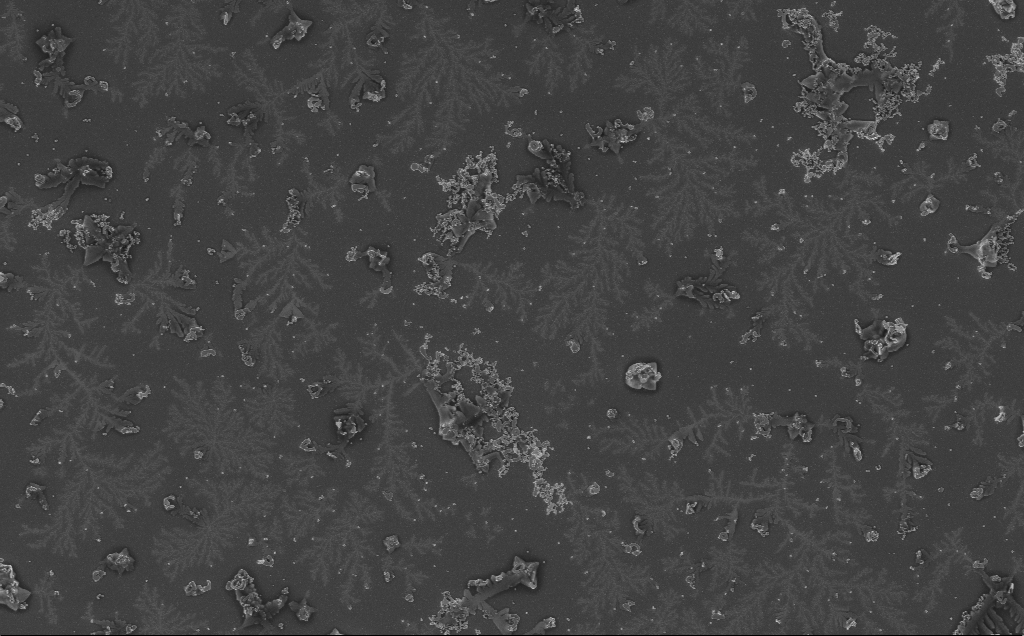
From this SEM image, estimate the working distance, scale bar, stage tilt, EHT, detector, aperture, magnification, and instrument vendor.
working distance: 3 mm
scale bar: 10000 nm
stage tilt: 0°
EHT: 5 kV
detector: InLens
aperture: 30 µm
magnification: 5 K X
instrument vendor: Zeiss